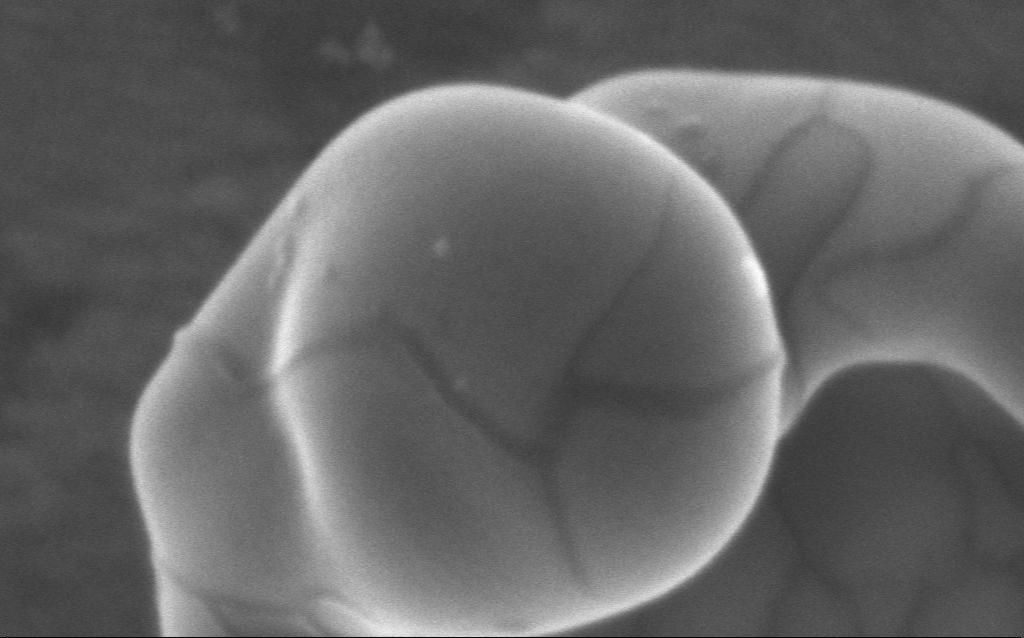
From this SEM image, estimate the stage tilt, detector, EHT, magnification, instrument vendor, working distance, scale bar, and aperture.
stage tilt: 0°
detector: InLens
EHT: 5 kV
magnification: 511.26 K X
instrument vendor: Zeiss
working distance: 4 mm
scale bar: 100 nm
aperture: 30 µm